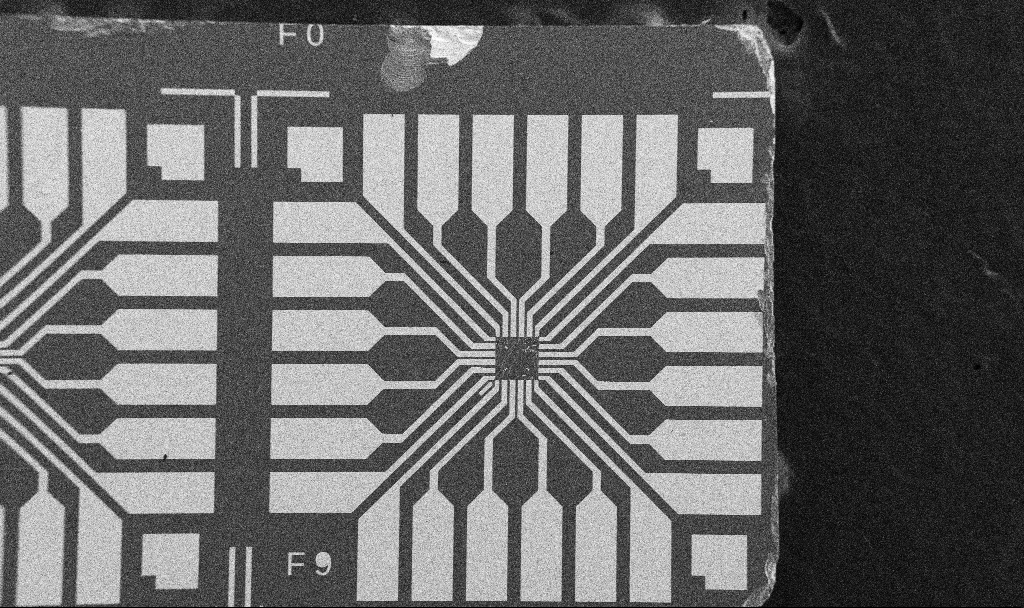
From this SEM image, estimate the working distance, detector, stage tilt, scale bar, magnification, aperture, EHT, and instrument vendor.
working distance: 10.7 mm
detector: SE2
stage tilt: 0°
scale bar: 200000 nm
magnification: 0.1 K X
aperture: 30 µm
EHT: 5 kV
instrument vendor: Zeiss